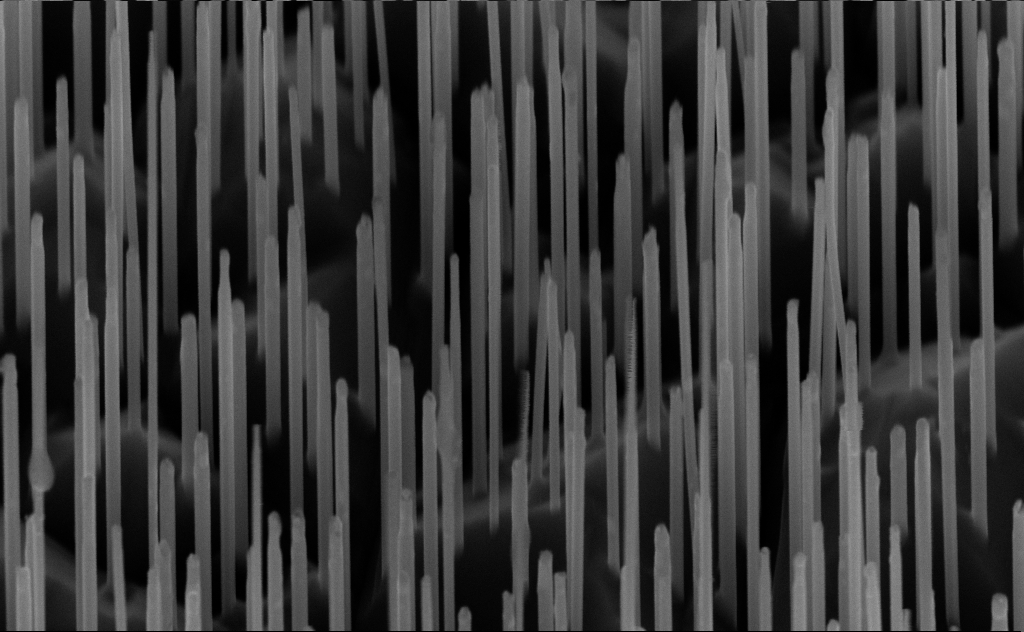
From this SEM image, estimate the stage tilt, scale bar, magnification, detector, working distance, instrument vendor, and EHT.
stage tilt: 45°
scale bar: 200 nm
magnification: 80 K X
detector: InLens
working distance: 6 mm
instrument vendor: Zeiss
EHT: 10 kV